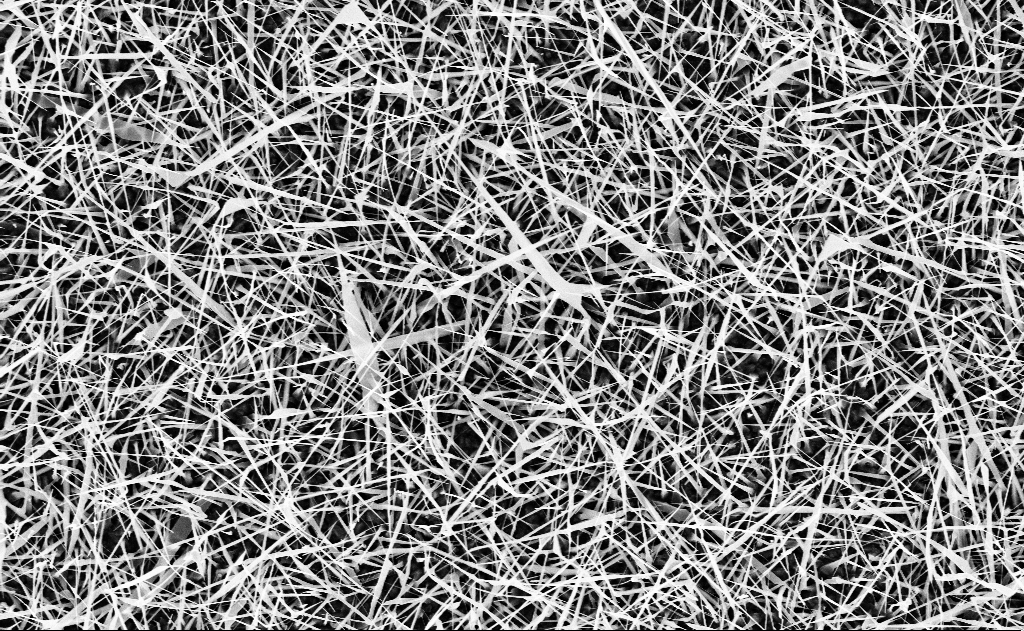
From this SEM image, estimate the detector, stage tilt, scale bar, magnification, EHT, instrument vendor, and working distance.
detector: InLens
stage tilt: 0°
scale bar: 2000 nm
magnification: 10 K X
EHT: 10 kV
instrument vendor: Zeiss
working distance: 15 mm